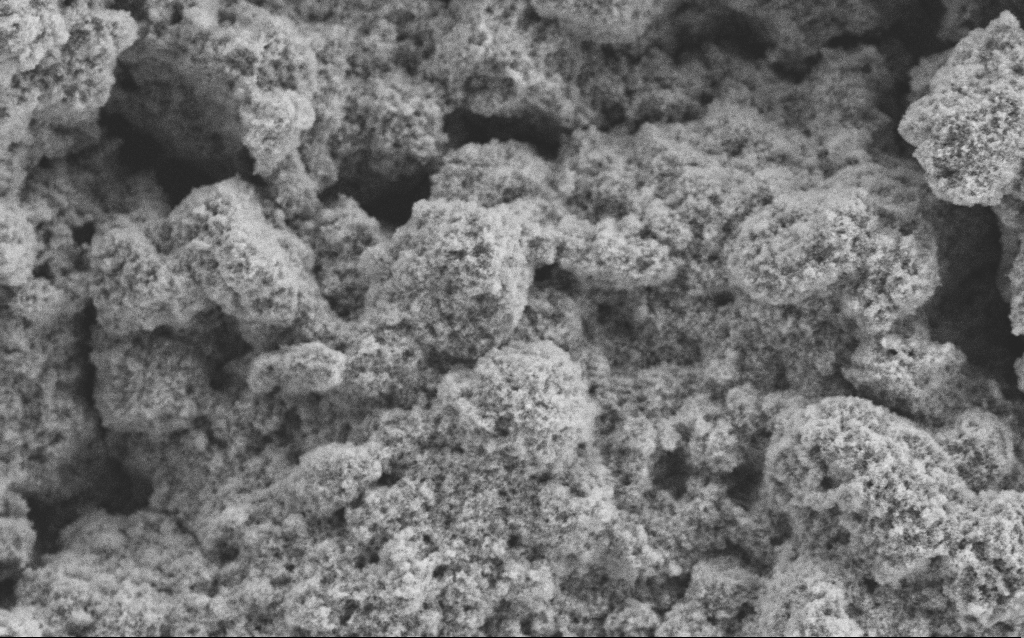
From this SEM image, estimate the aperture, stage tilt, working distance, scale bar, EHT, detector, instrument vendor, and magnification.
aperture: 30 µm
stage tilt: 0°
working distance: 4.1 mm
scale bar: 10000 nm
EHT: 5 kV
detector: SE2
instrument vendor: Zeiss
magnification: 6.41 K X